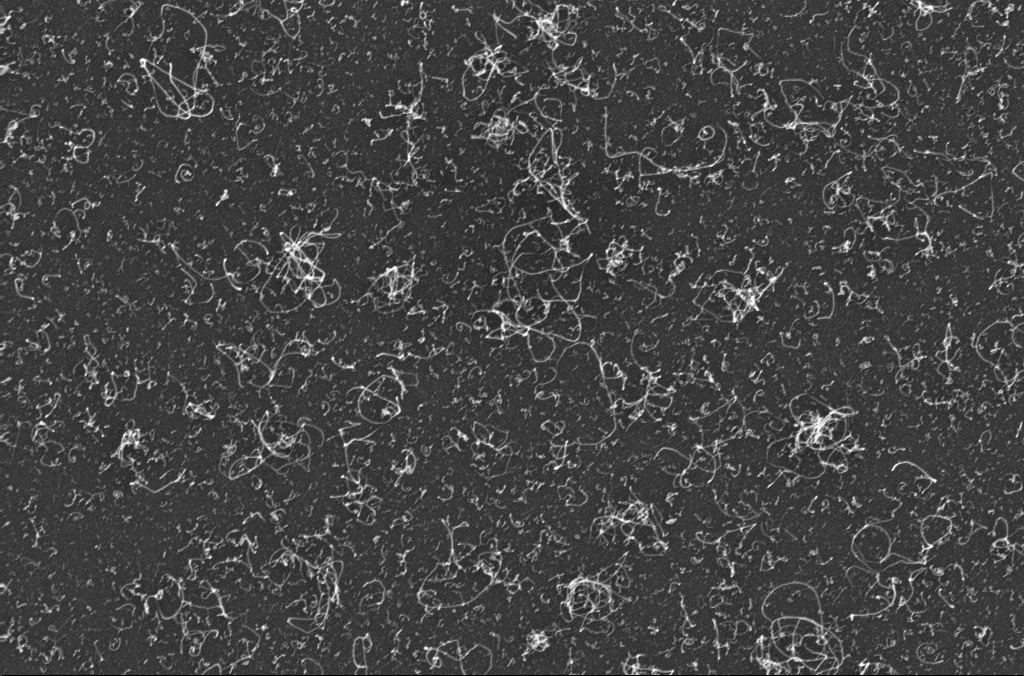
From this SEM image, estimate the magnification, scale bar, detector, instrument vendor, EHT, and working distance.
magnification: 50 K X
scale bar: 1000 nm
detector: InLens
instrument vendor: Zeiss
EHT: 10 kV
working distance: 3.3 mm